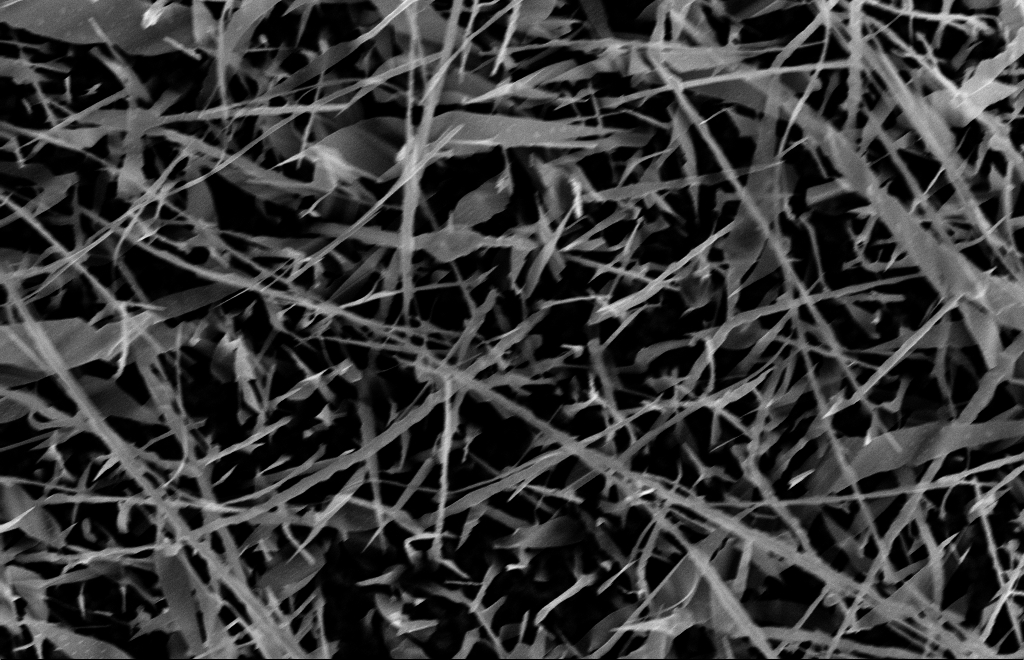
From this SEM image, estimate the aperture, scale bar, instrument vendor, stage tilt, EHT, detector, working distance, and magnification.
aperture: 30 µm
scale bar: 1000 nm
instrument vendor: Zeiss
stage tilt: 0°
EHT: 10 kV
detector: InLens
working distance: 15 mm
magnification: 20 K X